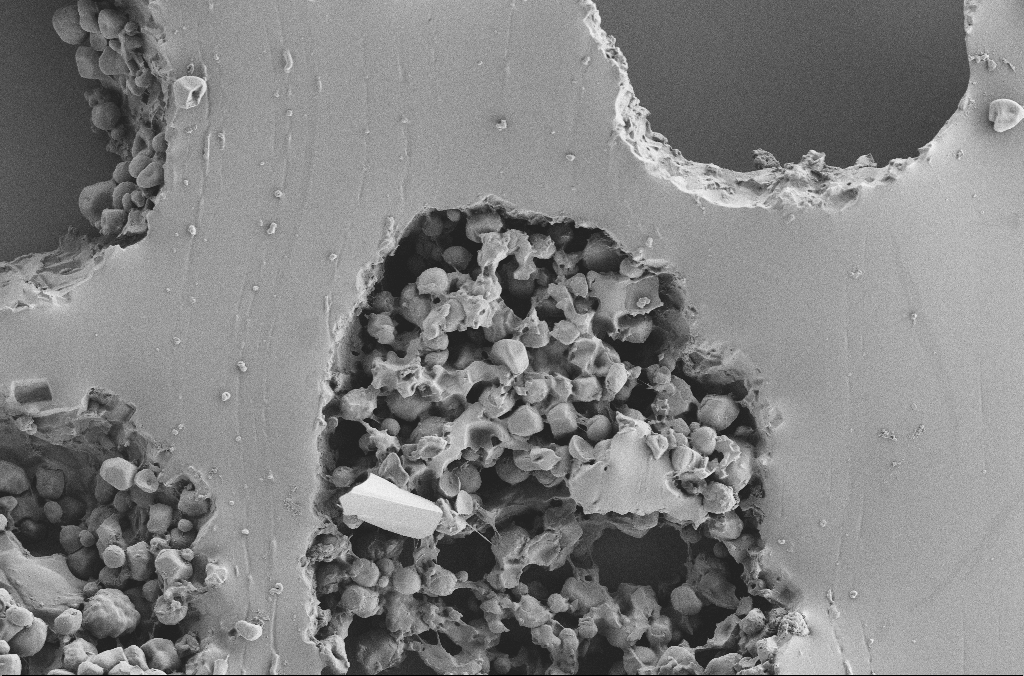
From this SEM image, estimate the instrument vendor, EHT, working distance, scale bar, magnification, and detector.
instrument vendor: Zeiss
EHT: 2 kV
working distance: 3.6 mm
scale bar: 20000 nm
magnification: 1 K X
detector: SE2